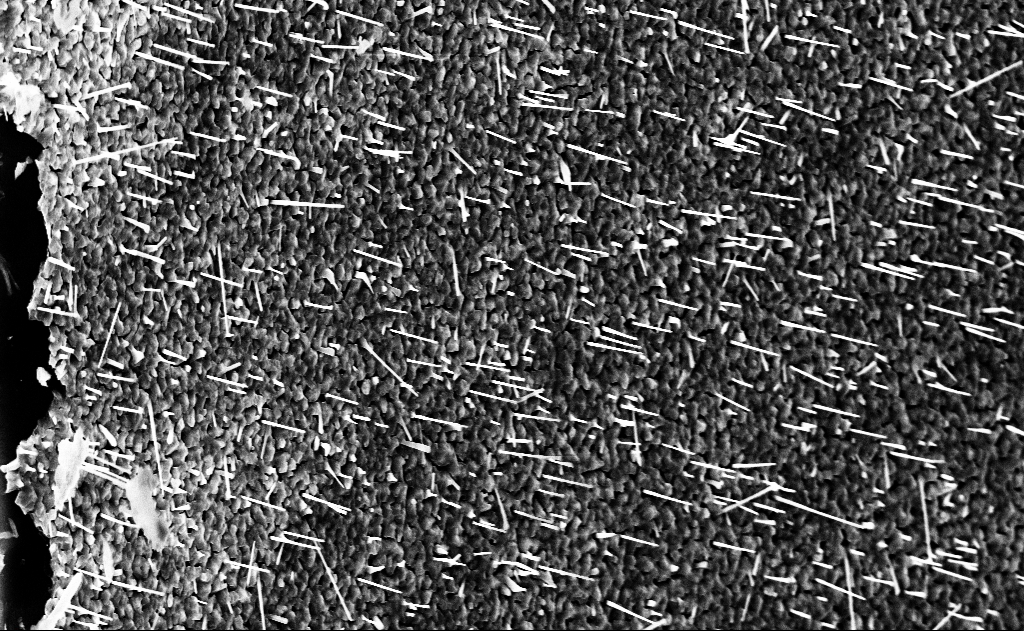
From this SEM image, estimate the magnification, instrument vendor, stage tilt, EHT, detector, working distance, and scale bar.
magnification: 10 K X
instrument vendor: Zeiss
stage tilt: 0°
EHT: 10 kV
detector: InLens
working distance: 14 mm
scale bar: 2000 nm